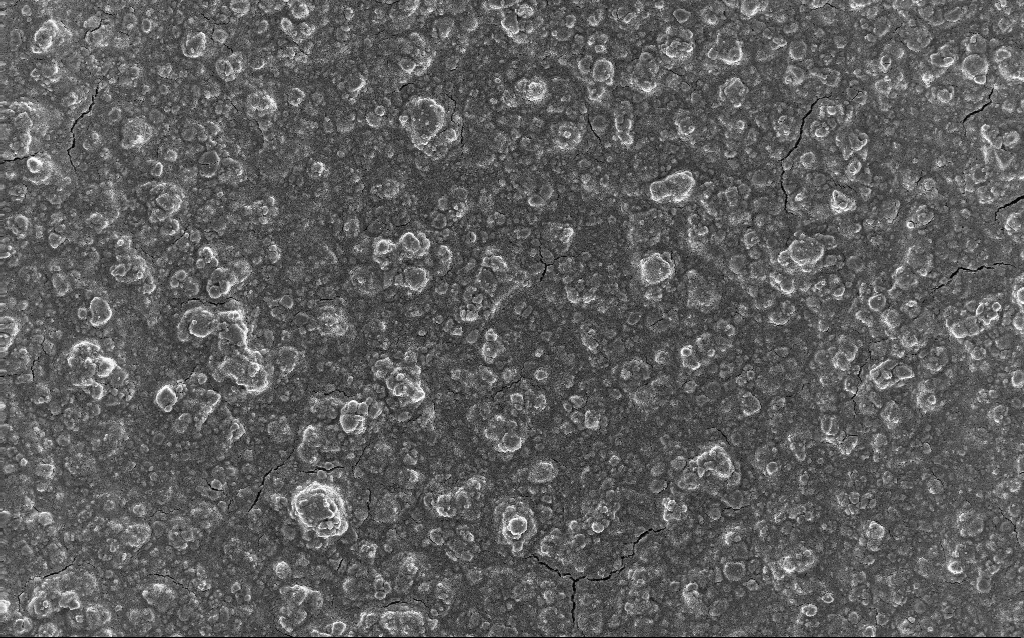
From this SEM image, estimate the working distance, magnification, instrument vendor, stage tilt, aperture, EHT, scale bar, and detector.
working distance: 2.7 mm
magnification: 0.77 K X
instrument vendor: Zeiss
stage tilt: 0°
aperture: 30 µm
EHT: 10 kV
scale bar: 20000 nm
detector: InLens